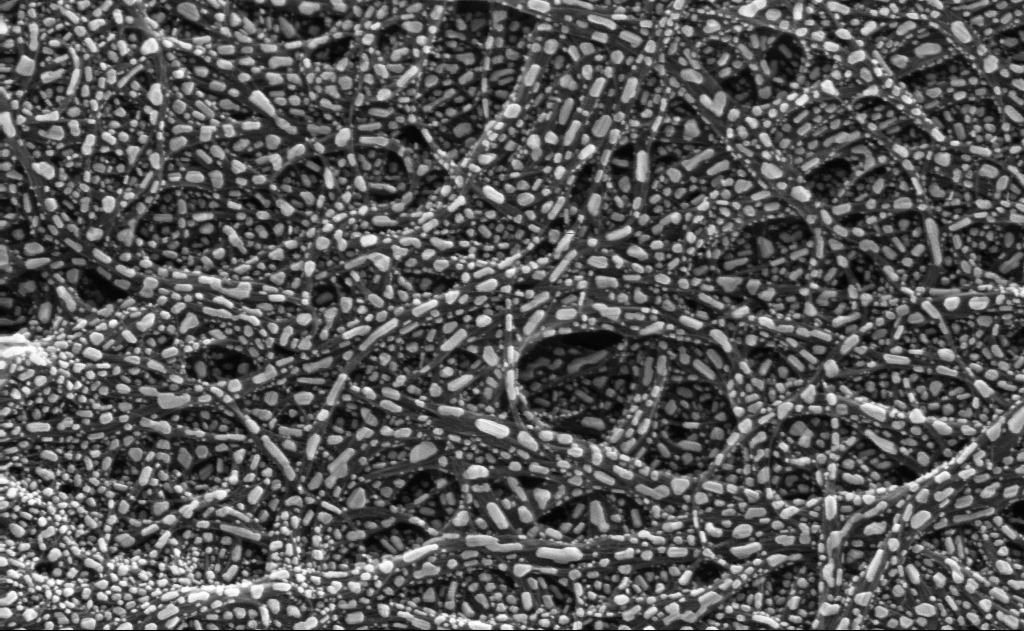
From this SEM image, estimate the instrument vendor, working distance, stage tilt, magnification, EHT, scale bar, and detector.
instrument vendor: Zeiss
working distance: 3 mm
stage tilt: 0°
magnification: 186.63 K X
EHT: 10 kV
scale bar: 100 nm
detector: InLens